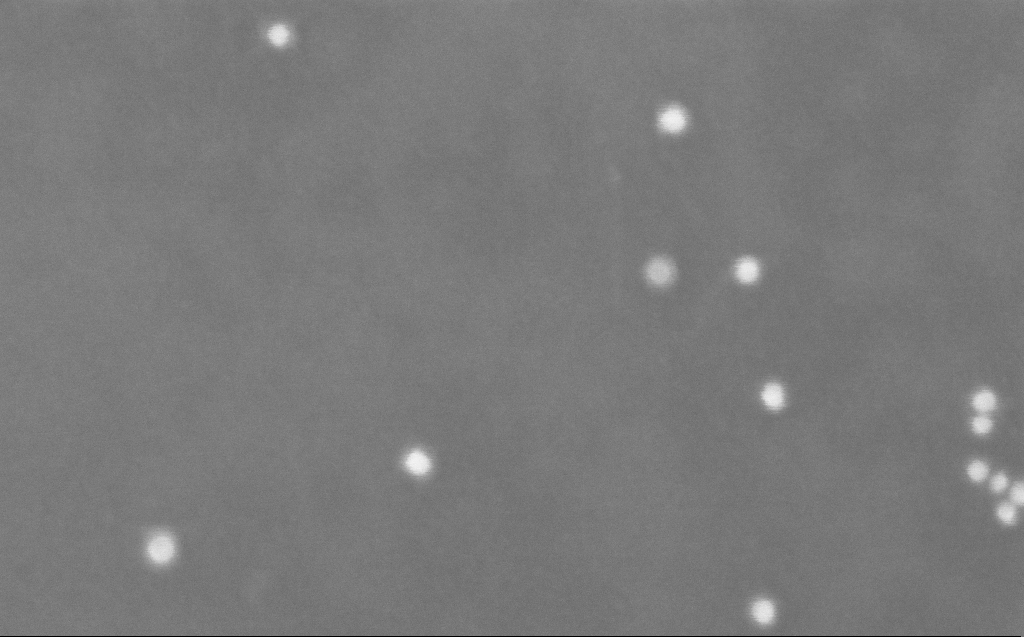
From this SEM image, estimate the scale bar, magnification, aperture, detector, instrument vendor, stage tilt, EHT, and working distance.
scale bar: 100 nm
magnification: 510.1 K X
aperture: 30 µm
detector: InLens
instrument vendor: Zeiss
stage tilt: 0°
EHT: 10 kV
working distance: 3 mm